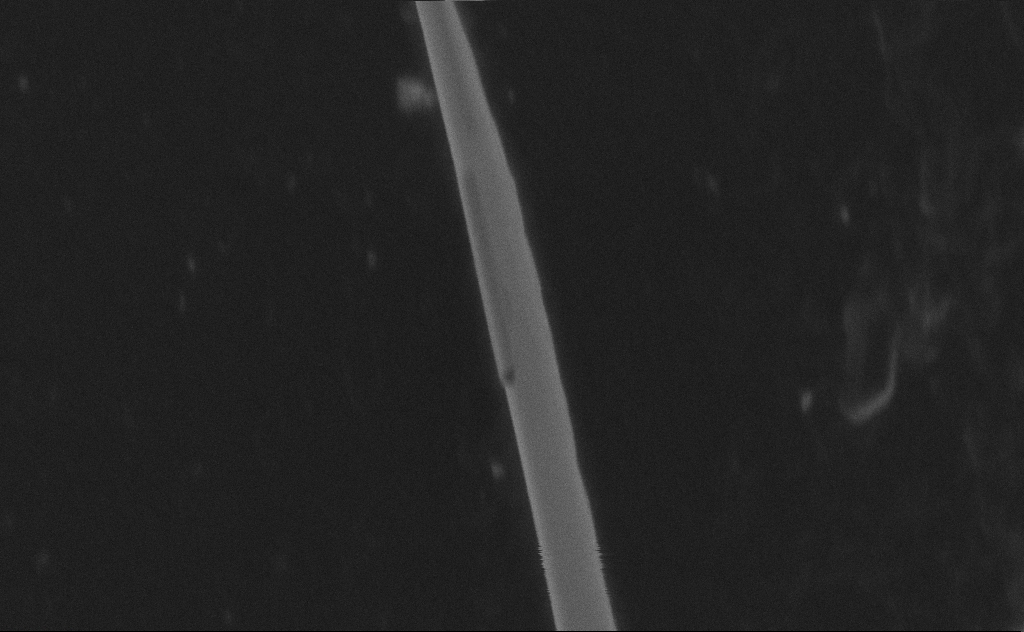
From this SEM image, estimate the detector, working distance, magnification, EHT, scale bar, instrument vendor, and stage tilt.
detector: SE2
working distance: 9 mm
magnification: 135.36 K X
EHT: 20 kV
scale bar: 200 nm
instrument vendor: Zeiss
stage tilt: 0°